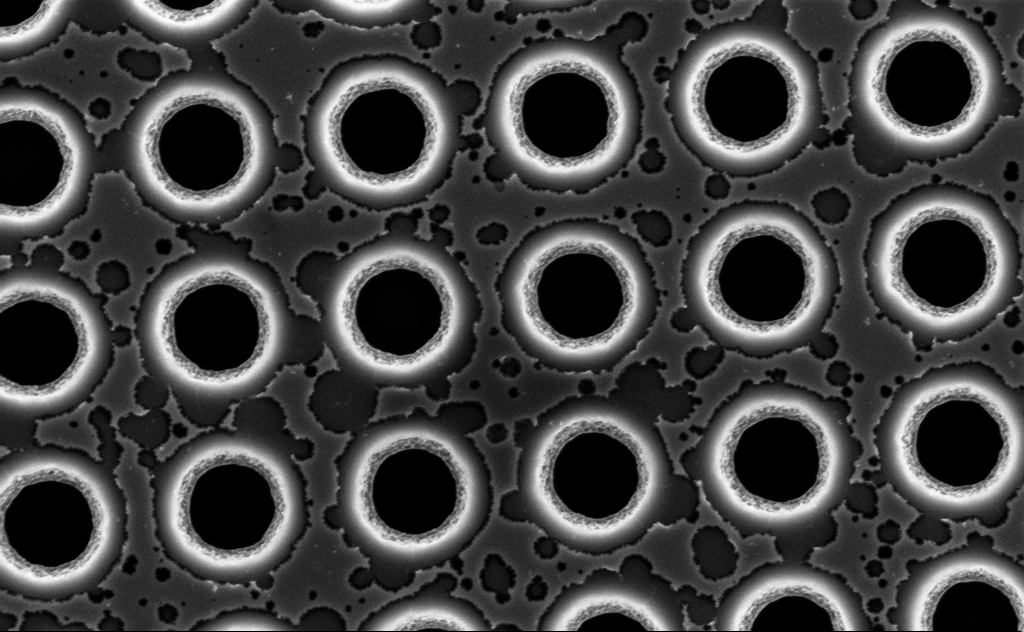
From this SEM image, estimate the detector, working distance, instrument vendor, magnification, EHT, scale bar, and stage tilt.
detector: InLens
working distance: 8 mm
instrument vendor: Zeiss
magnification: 63.65 K X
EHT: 3 kV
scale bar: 1000 nm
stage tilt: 0°